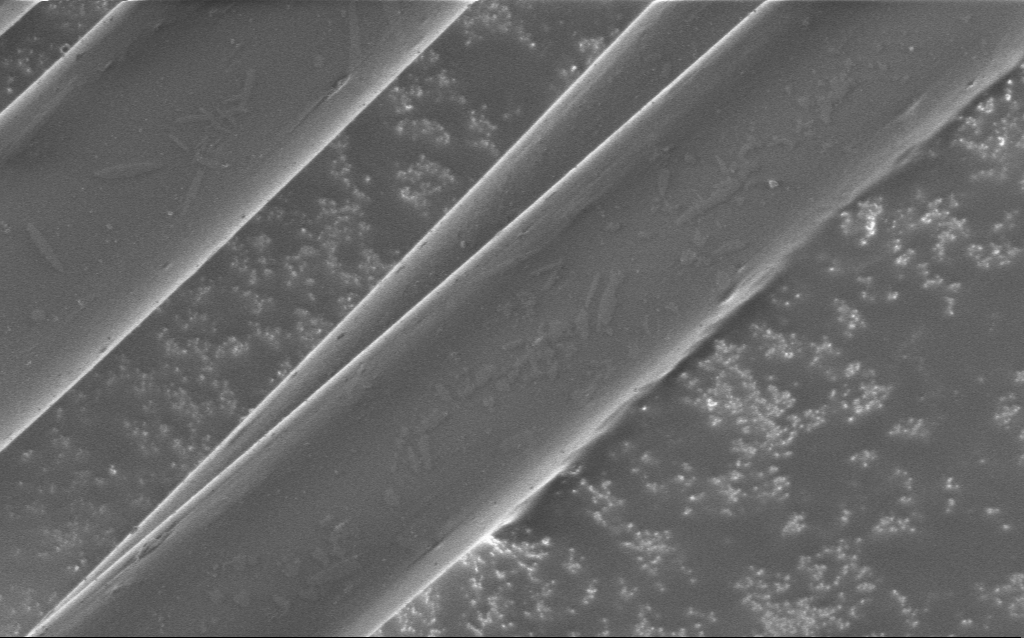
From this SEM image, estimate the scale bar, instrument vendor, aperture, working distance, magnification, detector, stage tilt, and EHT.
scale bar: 10000 nm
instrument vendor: Zeiss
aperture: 30 µm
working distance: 5 mm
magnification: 6.41 K X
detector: InLens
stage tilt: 0°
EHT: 1 kV